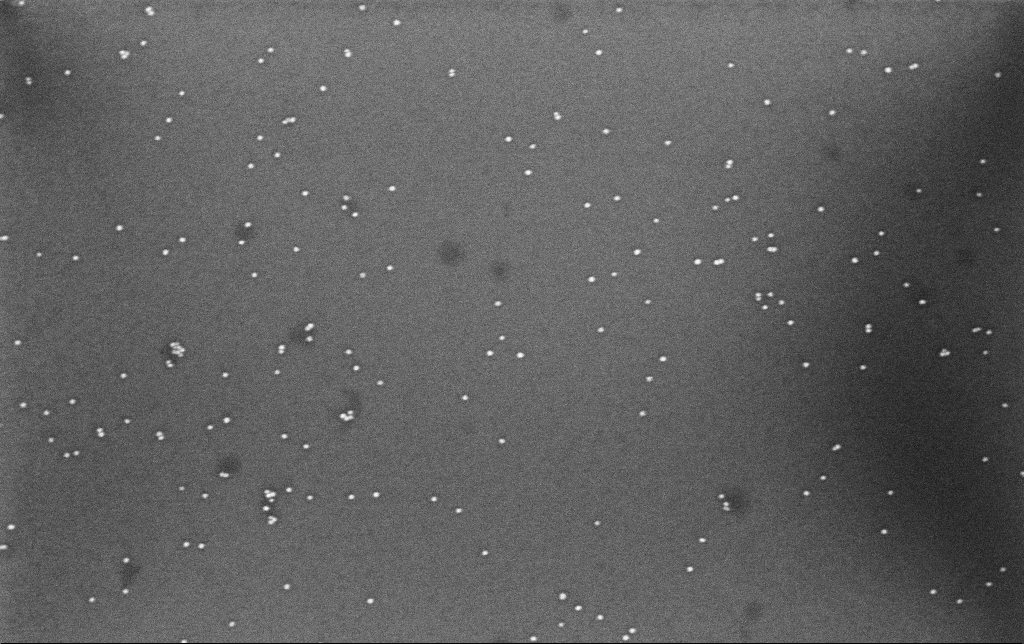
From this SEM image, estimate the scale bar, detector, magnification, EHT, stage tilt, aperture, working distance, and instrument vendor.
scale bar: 200 nm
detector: InLens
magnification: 100 K X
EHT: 8 kV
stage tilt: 0°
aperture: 30 µm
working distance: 6.6 mm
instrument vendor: Zeiss